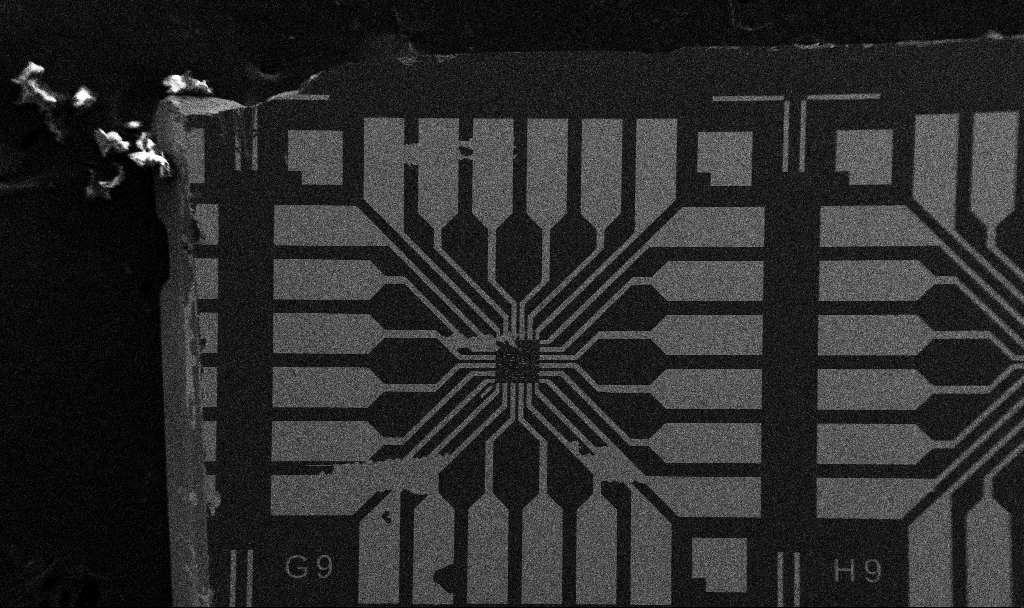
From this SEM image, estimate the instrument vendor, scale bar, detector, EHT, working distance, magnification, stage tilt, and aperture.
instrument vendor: Zeiss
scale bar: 200000 nm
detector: SE2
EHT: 5 kV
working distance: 10.7 mm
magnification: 0.1 K X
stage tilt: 0°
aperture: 30 µm